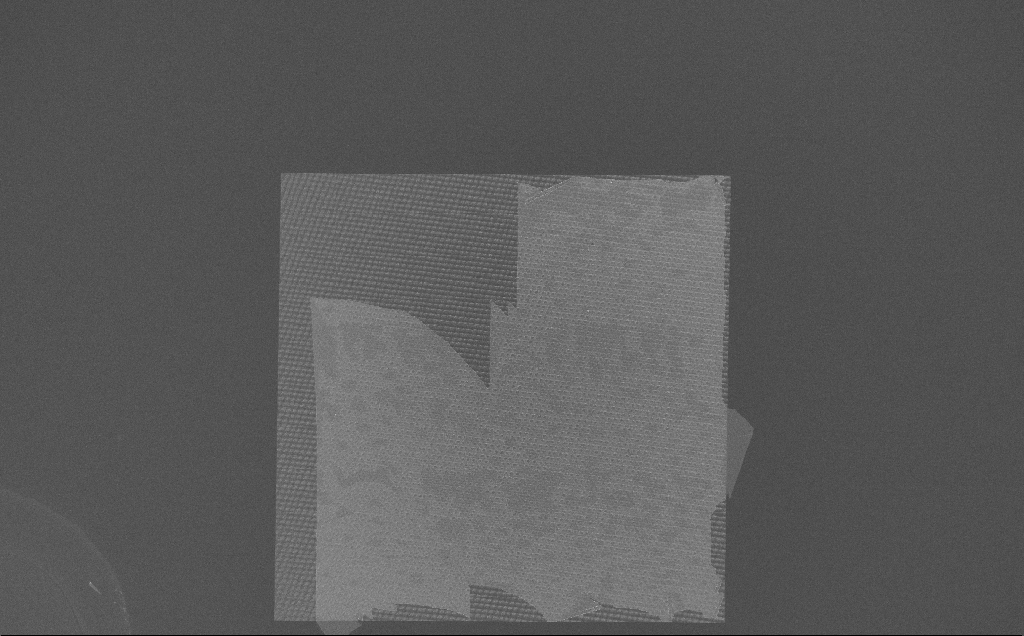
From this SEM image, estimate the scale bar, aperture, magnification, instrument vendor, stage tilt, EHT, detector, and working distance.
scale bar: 100000 nm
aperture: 30 µm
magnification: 0.222 K X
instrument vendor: Zeiss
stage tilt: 0°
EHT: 10 kV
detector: InLens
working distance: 7 mm